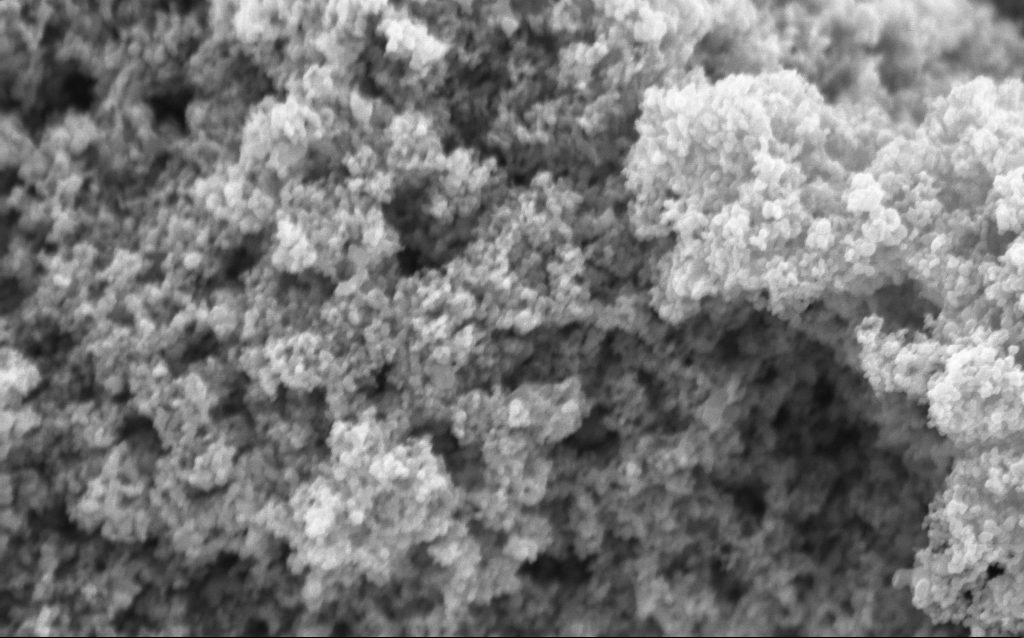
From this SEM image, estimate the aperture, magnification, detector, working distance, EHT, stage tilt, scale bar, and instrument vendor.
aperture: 30 µm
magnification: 114.61 K X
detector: InLens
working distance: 4.6 mm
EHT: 5 kV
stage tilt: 0°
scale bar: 200 nm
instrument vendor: Zeiss